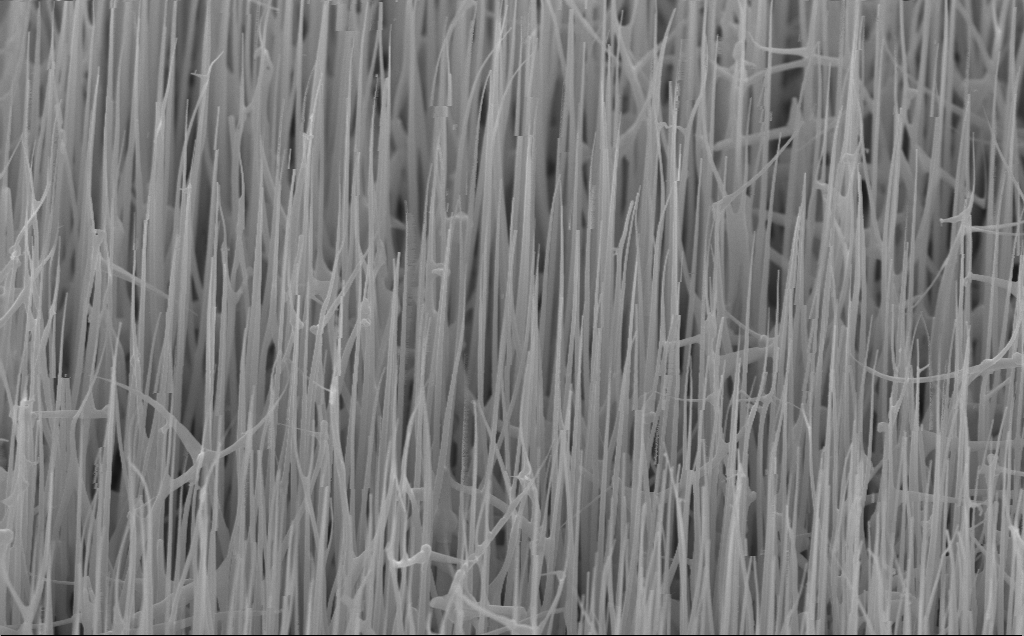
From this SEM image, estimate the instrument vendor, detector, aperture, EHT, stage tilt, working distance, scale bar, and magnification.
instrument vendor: Zeiss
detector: InLens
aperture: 30 µm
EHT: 10 kV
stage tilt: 45°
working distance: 6 mm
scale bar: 1000 nm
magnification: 40 K X